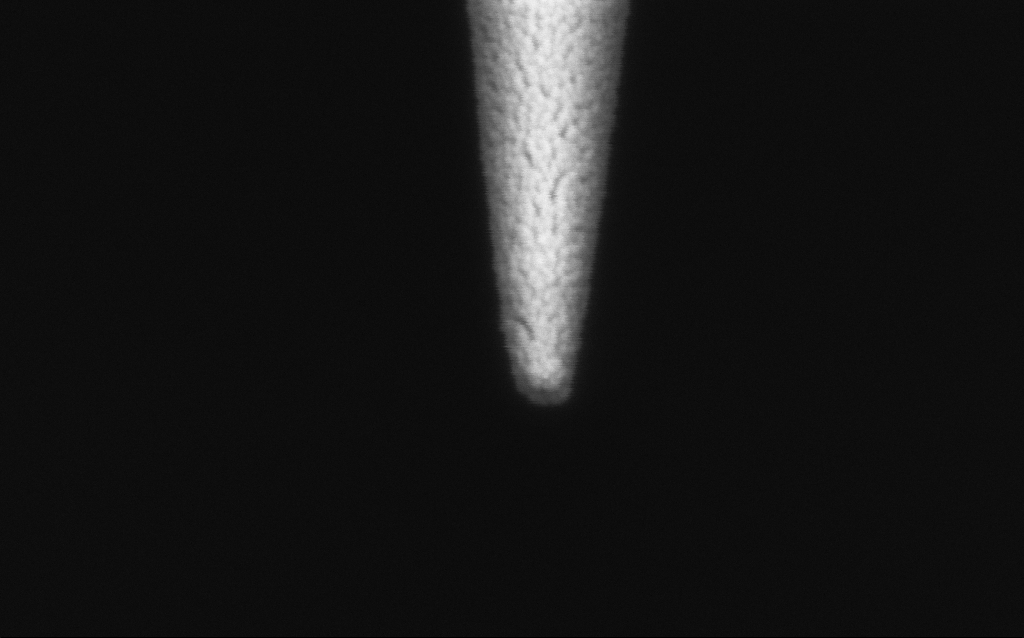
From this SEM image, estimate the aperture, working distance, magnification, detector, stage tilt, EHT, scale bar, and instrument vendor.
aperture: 30 µm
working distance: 7.8 mm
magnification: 250 K X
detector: InLens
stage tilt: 45°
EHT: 2 kV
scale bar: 200 nm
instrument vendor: Zeiss